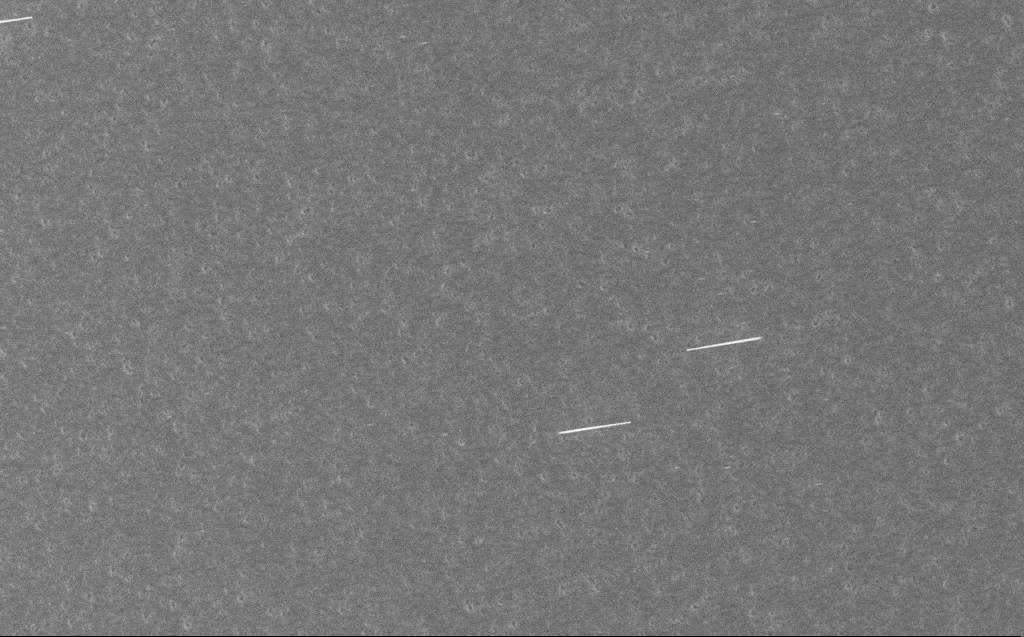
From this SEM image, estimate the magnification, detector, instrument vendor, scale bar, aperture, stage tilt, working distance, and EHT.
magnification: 5 K X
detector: InLens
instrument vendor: Zeiss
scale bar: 10000 nm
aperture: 30 µm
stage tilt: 0°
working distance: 3 mm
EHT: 10 kV